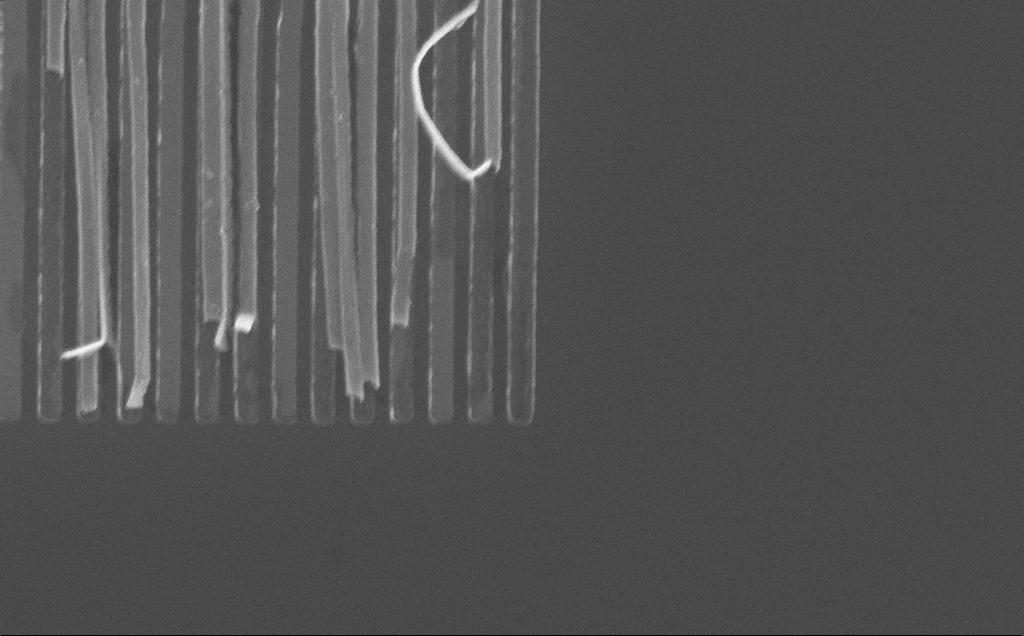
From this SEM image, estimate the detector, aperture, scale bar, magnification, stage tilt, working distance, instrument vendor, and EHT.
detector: InLens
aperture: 30 µm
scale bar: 1000 nm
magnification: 57.52 K X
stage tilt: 0°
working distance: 7 mm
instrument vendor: Zeiss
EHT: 10 kV